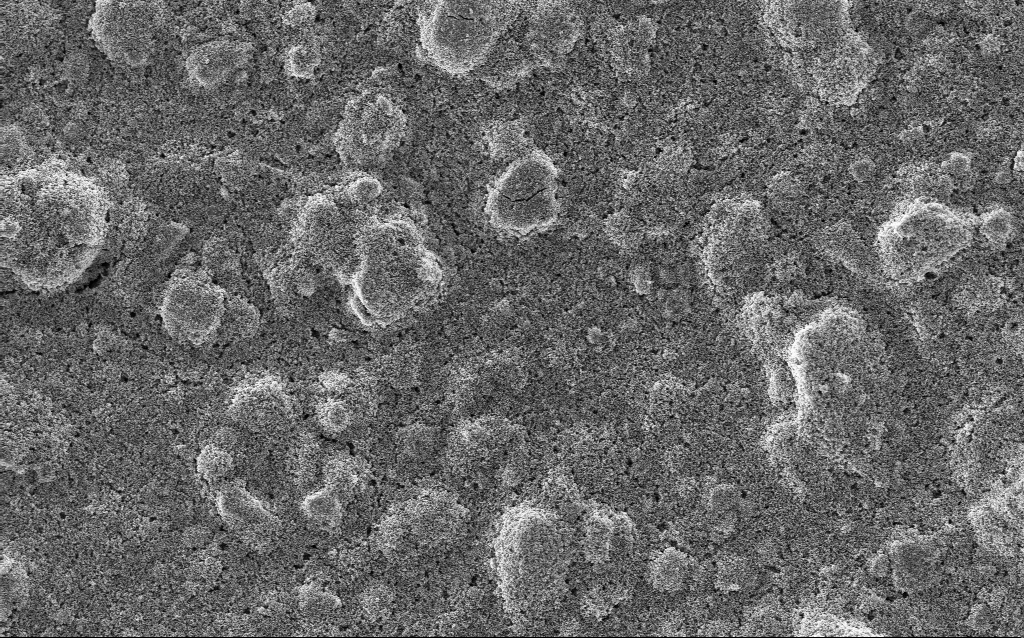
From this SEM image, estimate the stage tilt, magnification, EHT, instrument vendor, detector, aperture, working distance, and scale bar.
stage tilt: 0°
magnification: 5 K X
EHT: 5 kV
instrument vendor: Zeiss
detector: InLens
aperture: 30 µm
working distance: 2.9 mm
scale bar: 10000 nm